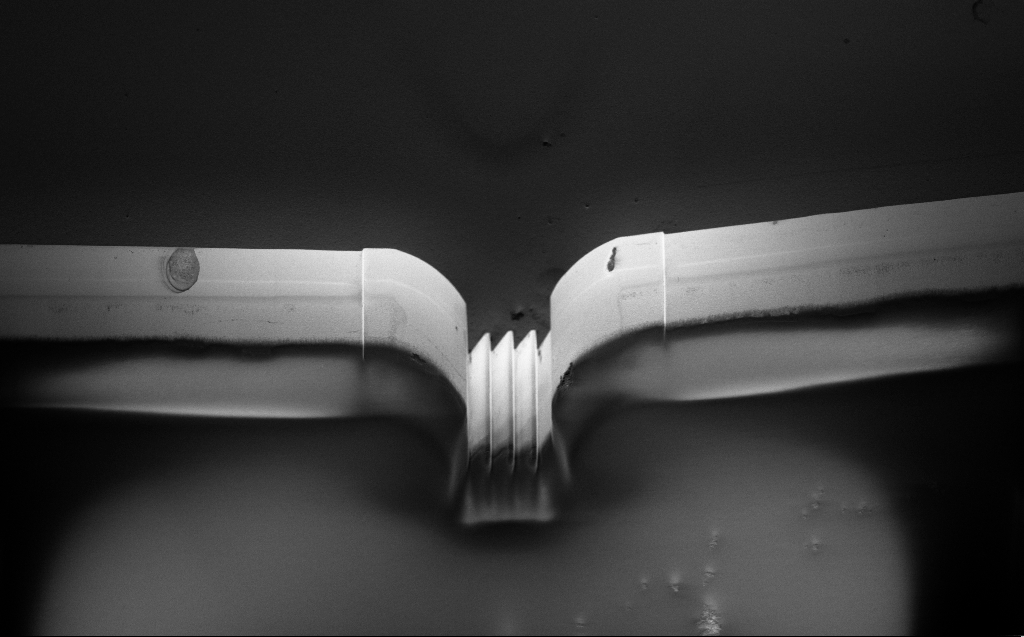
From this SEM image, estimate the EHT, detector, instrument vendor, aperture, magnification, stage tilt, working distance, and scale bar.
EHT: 1 kV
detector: InLens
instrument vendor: Zeiss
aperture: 30 µm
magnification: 0.749 K X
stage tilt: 45°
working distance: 6 mm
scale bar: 20000 nm